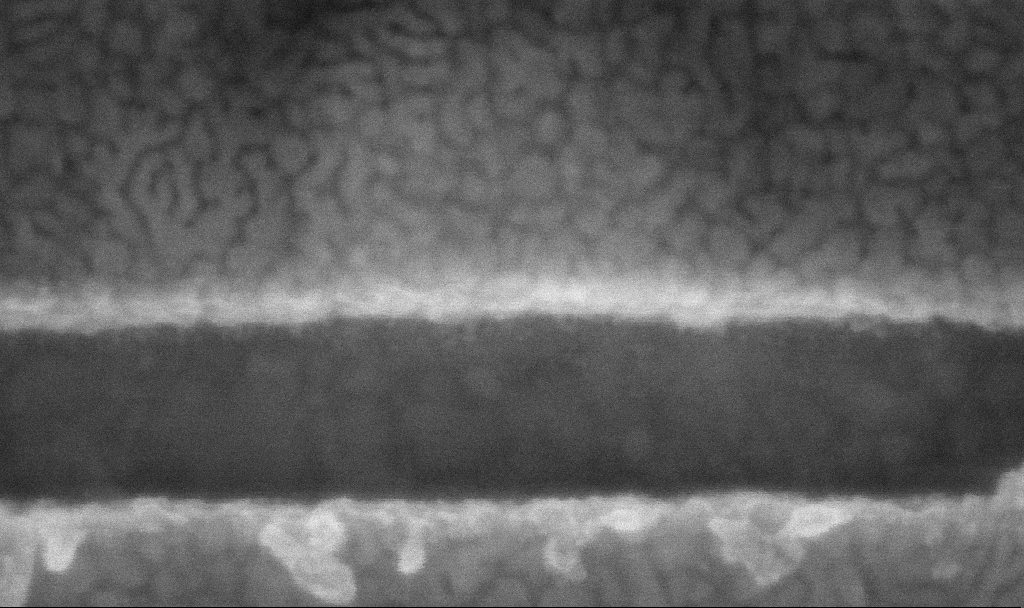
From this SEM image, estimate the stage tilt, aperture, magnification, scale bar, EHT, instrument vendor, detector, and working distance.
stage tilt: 0°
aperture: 60 µm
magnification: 543.07 K X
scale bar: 100 nm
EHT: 5 kV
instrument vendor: Zeiss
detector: InLens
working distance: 3.8 mm